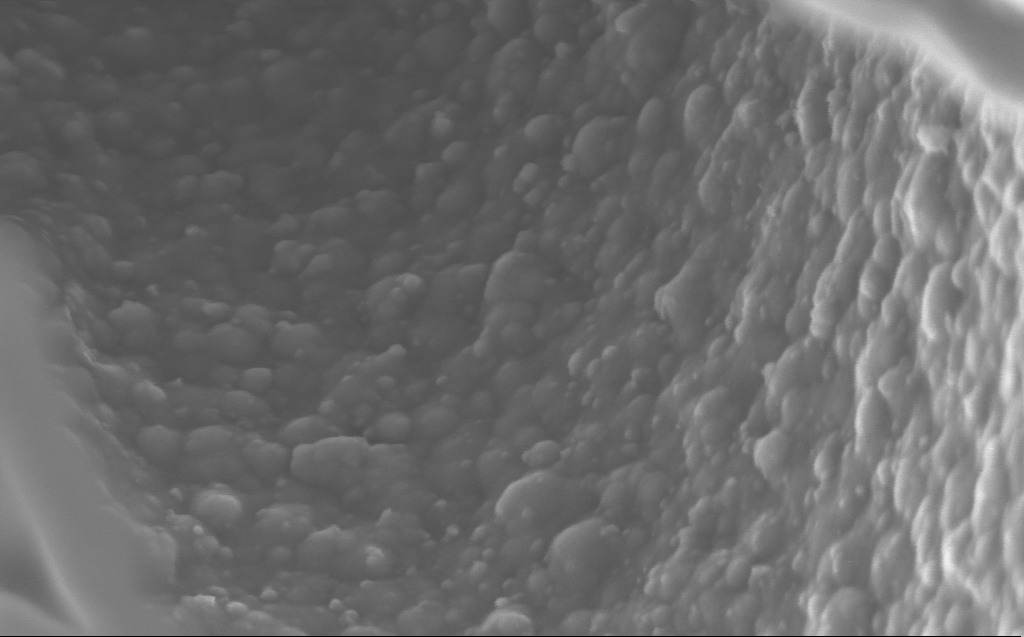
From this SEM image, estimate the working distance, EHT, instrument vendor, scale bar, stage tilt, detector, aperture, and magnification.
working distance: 2 mm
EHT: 2 kV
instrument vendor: Zeiss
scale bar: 200 nm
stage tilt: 45°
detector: InLens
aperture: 30 µm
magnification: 218.16 K X